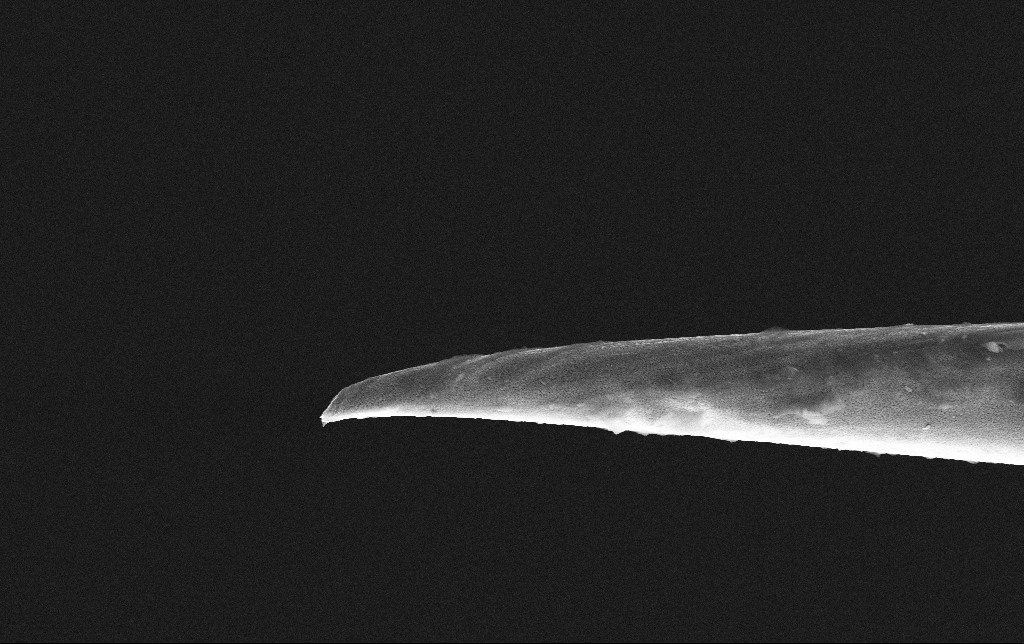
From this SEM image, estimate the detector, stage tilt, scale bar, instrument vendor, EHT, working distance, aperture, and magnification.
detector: SE2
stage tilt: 0°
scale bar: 2000 nm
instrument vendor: Zeiss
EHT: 10 kV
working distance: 8.7 mm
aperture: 30 µm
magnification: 8.98 K X